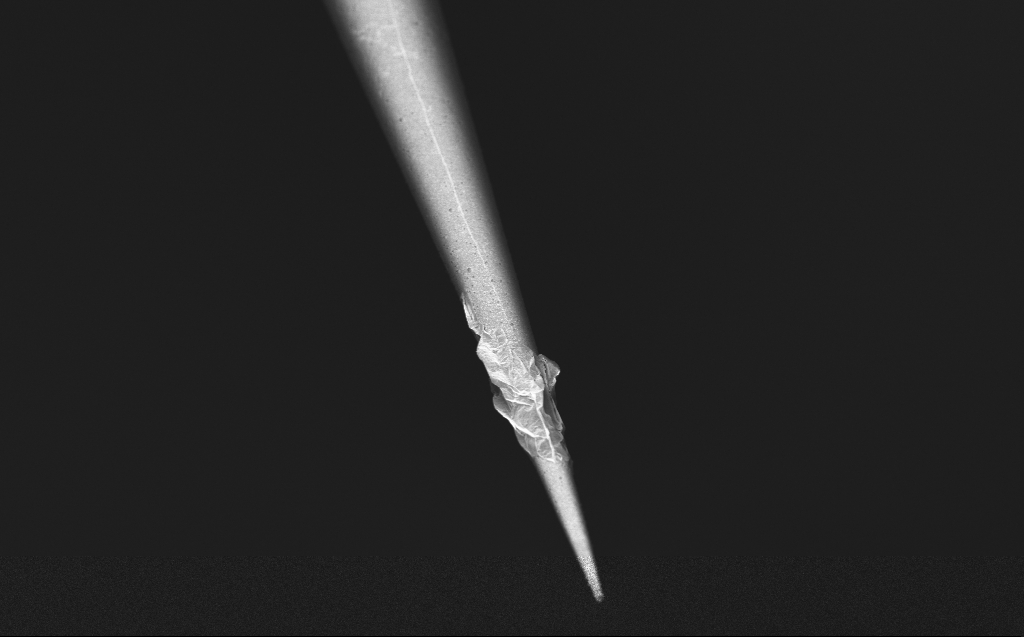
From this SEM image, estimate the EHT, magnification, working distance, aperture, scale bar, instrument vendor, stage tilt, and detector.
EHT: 1 kV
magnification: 5 K X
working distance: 4 mm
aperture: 30 µm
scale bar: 10000 nm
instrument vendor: Zeiss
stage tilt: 45°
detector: InLens